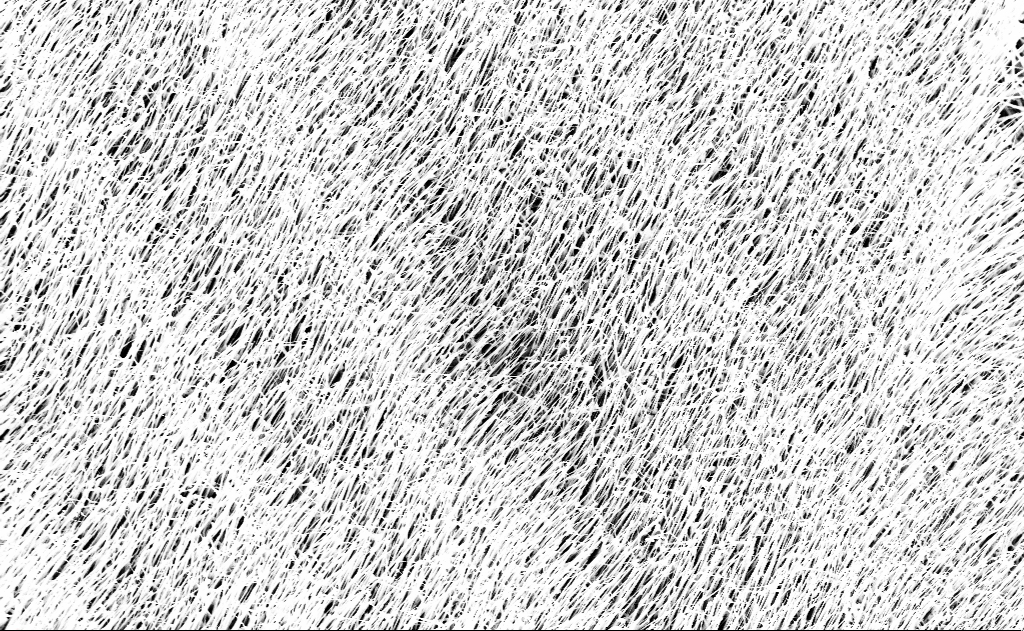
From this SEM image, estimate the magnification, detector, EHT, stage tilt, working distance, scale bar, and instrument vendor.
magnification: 10 K X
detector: InLens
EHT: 10 kV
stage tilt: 0°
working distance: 13 mm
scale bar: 2000 nm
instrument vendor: Zeiss